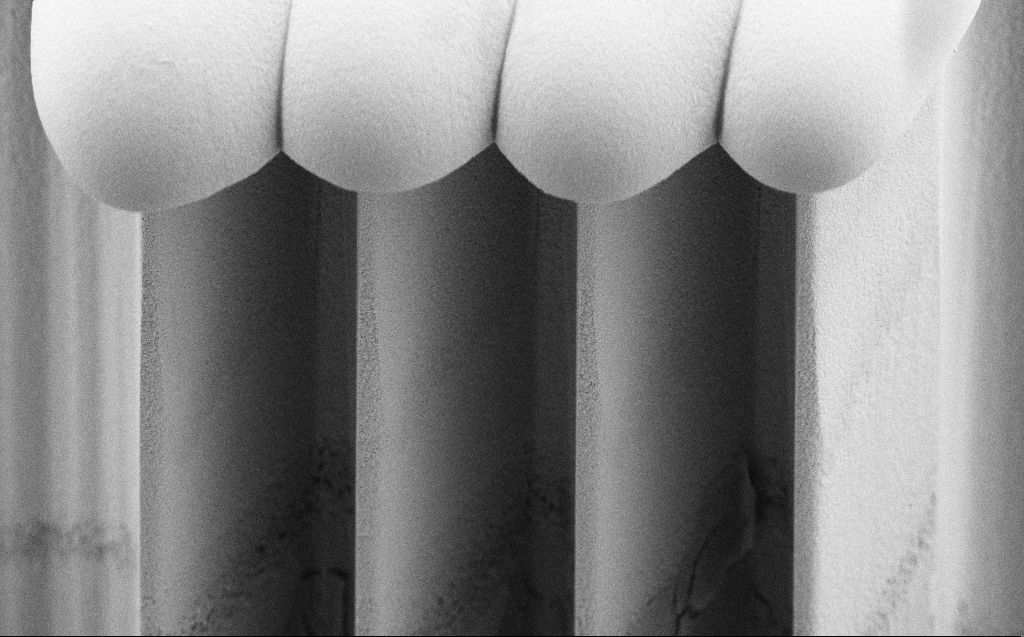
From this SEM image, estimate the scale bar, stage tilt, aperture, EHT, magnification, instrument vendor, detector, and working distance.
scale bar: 10000 nm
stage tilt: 45°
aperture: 30 µm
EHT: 5 kV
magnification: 7.08 K X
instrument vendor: Zeiss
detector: SE2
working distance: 5 mm